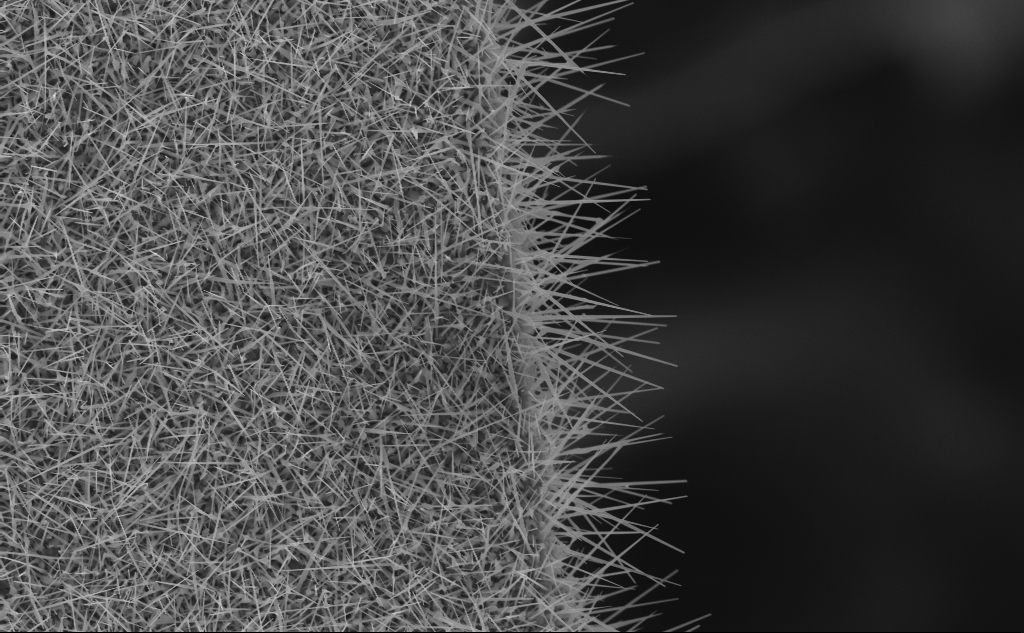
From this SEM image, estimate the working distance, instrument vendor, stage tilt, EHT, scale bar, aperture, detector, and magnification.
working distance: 7 mm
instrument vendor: Zeiss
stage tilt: -0.2°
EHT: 10 kV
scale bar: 2000 nm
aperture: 30 µm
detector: InLens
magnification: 10 K X